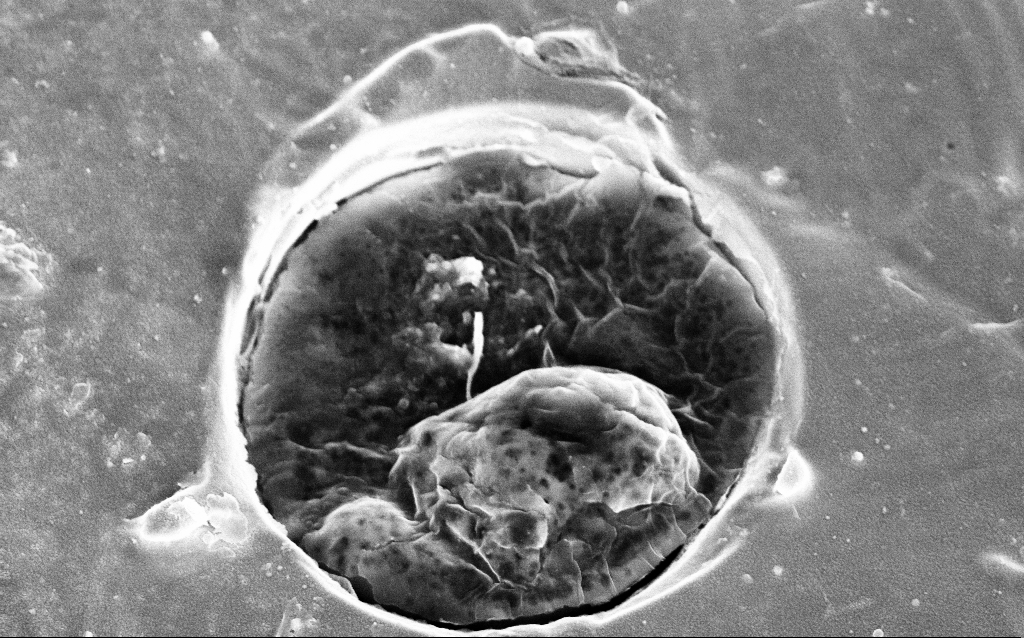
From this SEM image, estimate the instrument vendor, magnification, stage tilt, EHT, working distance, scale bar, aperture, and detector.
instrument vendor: Zeiss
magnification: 38.08 K X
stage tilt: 25.7°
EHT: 5 kV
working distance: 6.9 mm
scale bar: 1000 nm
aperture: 30 µm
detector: InLens